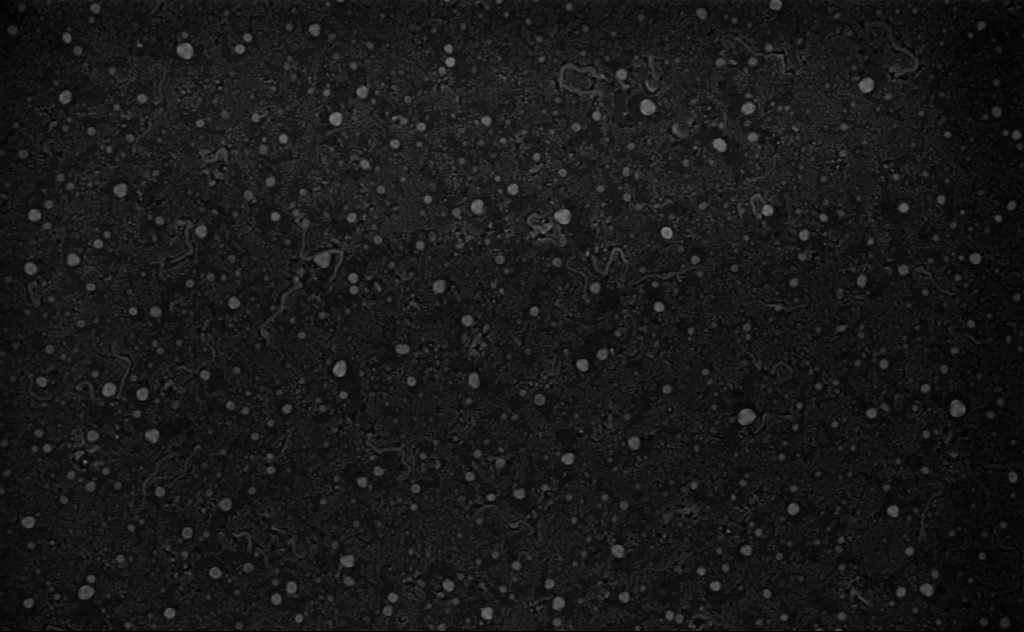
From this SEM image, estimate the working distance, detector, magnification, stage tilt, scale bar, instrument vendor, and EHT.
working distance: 4 mm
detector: InLens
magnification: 80 K X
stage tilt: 0°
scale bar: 200 nm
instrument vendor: Zeiss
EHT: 3 kV